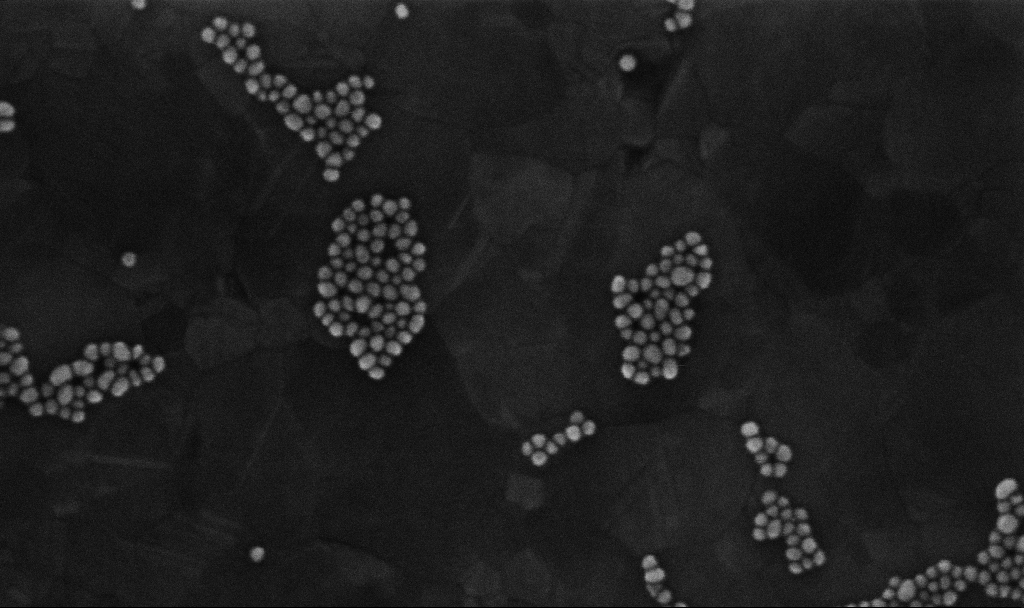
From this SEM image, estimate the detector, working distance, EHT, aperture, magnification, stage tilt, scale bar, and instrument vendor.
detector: InLens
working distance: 3.4 mm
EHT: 10 kV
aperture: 30 µm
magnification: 300 K X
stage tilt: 0°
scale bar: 200 nm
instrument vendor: Zeiss